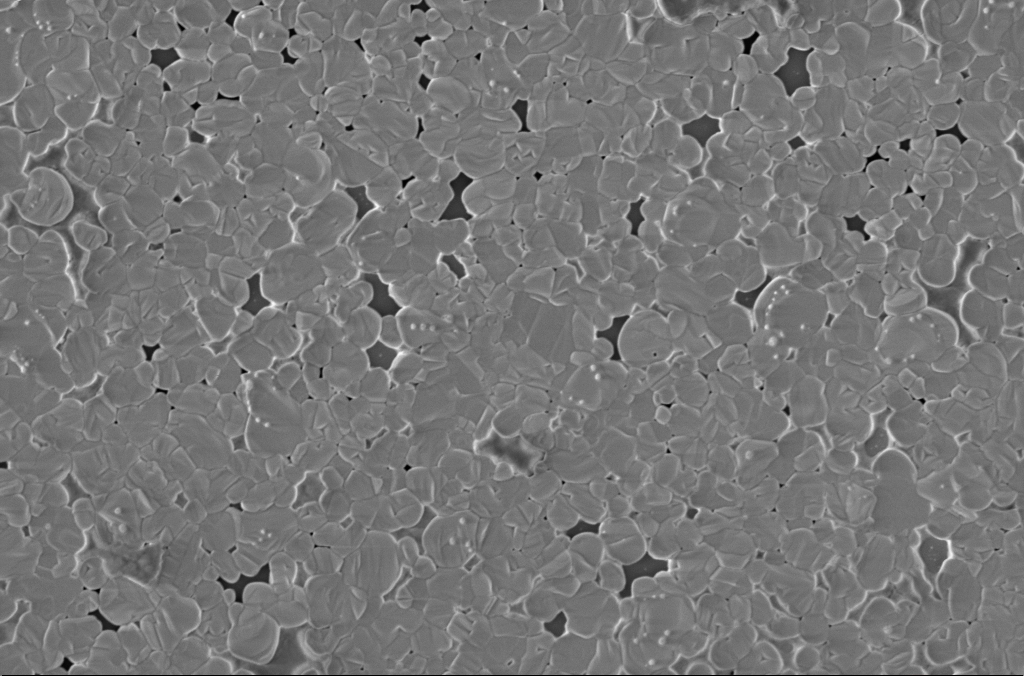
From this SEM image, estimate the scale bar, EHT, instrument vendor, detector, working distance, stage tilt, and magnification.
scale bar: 2000 nm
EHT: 2 kV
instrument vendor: Zeiss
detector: InLens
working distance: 3 mm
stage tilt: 0°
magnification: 20 K X